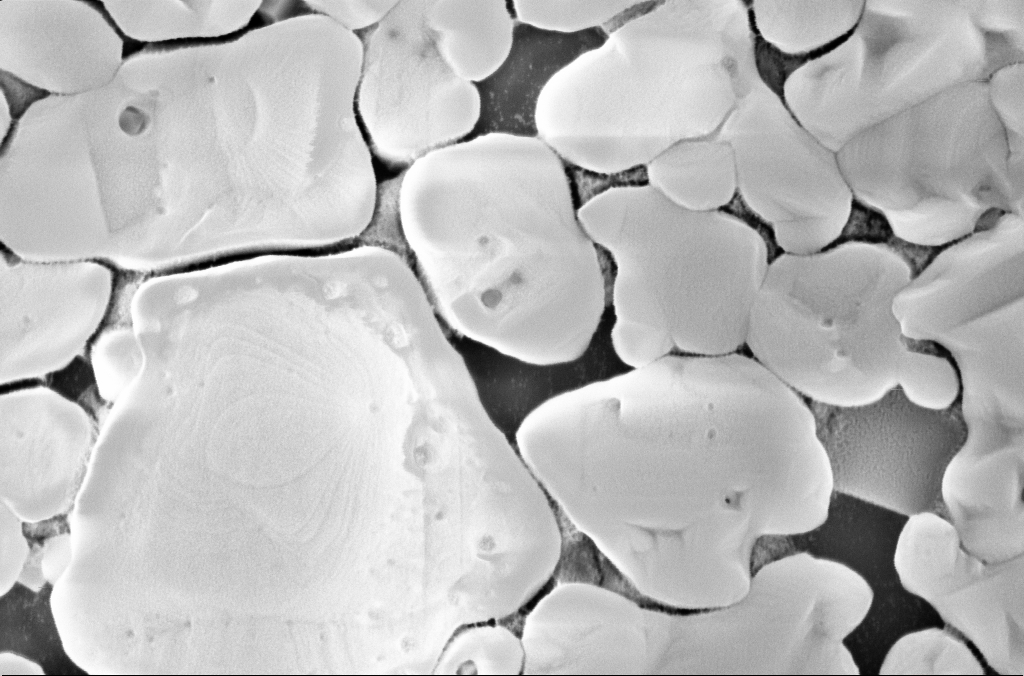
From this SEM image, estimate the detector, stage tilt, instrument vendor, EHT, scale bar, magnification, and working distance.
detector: InLens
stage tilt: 0°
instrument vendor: Zeiss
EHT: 5 kV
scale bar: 200 nm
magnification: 100 K X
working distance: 3 mm